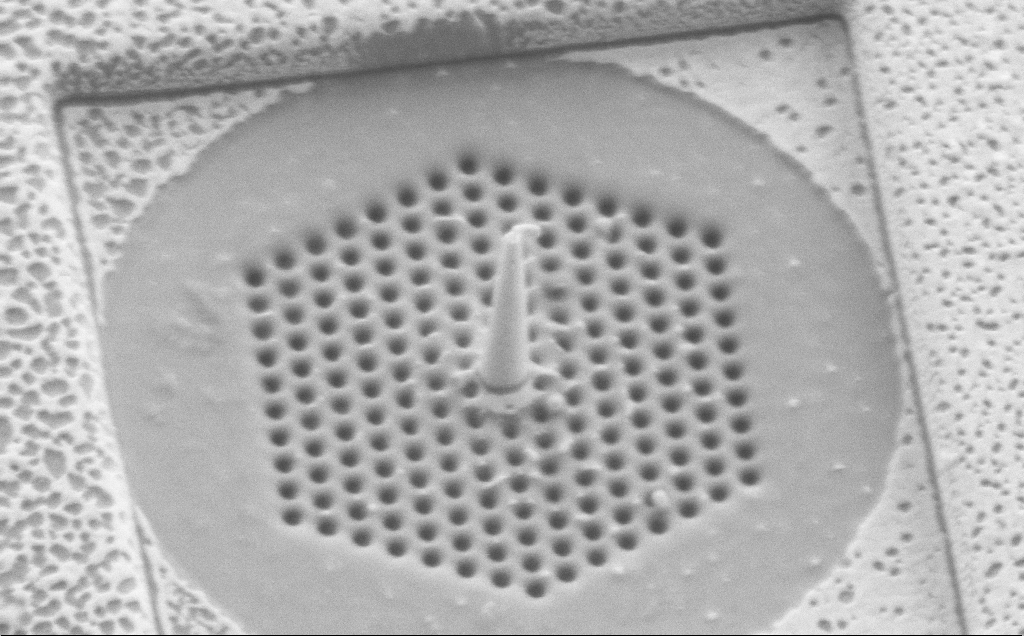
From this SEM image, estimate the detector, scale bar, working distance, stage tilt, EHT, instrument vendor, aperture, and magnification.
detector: SE2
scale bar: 1000 nm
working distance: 6 mm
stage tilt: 34.3°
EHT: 3 kV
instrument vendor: Zeiss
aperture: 30 µm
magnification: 48.67 K X